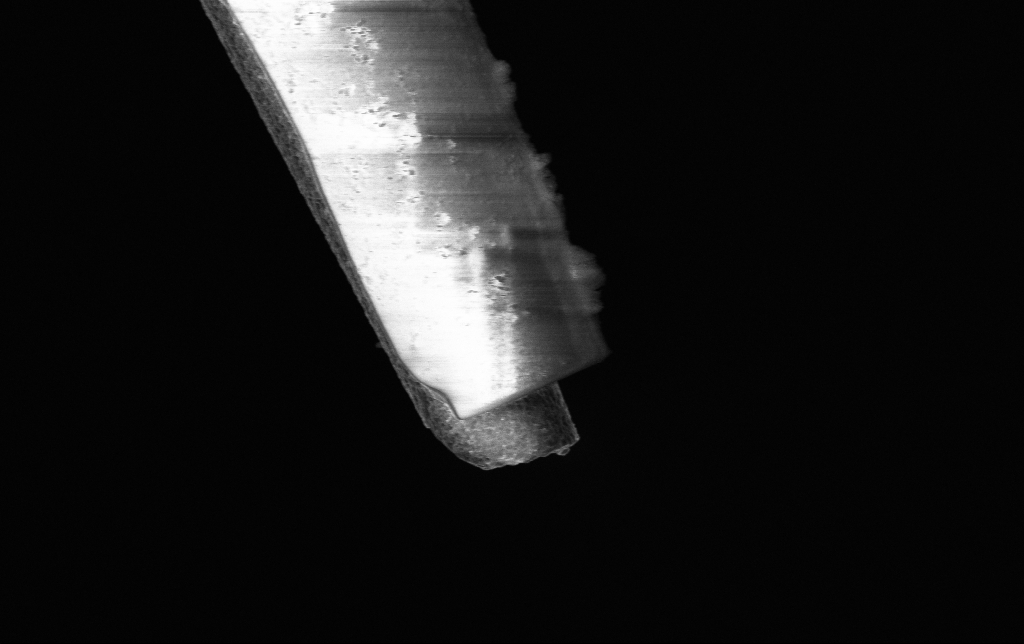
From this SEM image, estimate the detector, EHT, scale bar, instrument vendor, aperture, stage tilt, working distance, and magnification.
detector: InLens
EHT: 3 kV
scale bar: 2000 nm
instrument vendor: Zeiss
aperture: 30 µm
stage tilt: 0°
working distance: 6.5 mm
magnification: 20 K X